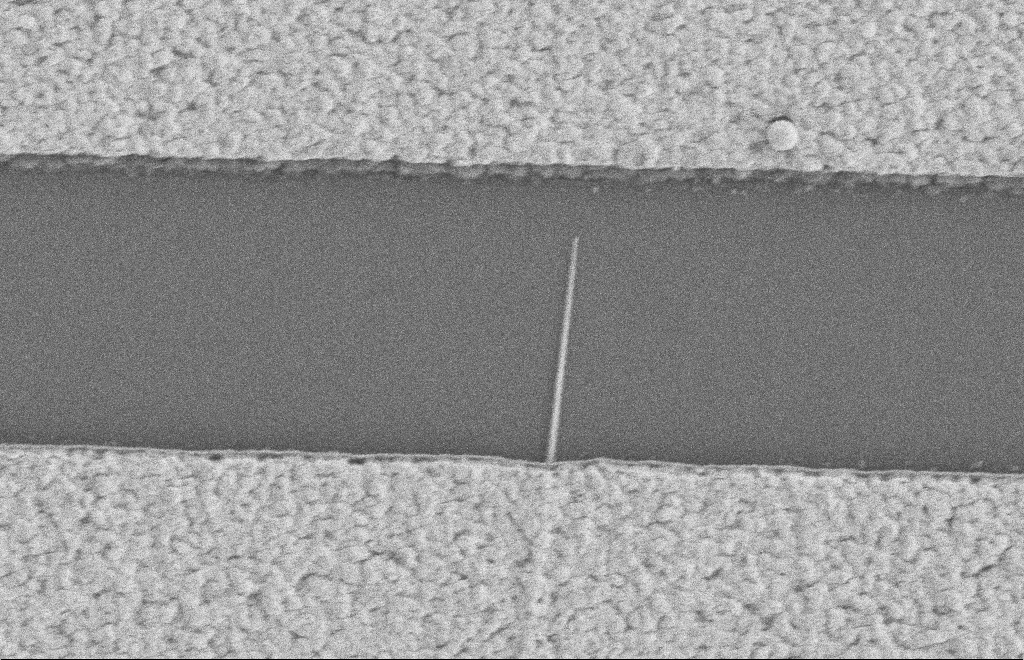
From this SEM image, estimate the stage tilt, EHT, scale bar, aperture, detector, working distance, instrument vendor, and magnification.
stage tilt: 0°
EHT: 2 kV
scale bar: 1000 nm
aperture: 20 µm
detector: SE2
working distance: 8 mm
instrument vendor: Zeiss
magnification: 38.56 K X